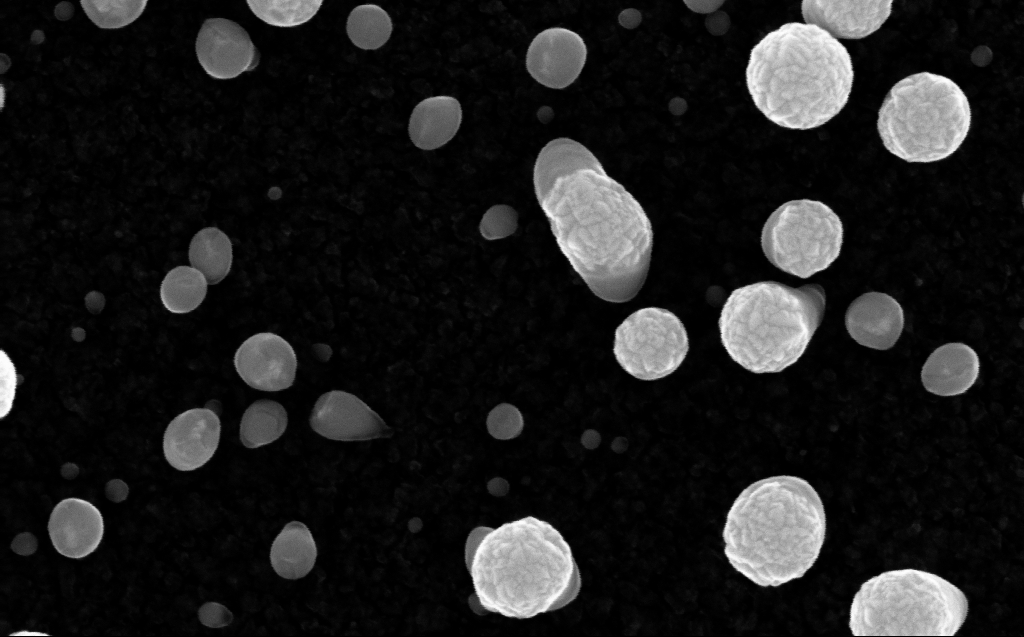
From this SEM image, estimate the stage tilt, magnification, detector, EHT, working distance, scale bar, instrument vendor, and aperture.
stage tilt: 0°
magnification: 200 K X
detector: InLens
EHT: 10 kV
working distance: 3 mm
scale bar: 100 nm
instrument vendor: Zeiss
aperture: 30 µm